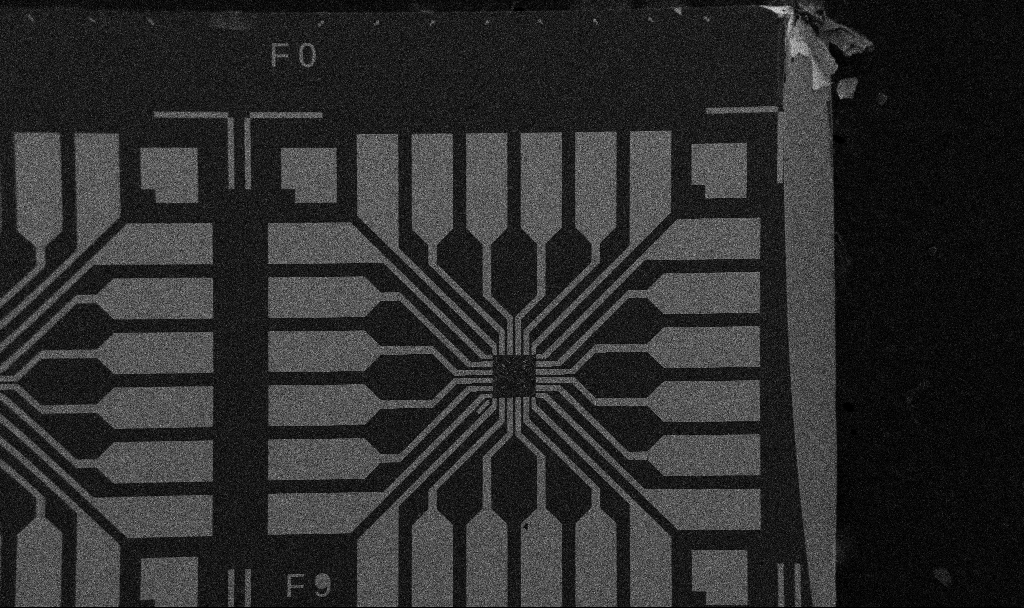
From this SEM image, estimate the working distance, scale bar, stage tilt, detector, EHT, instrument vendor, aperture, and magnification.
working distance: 10.7 mm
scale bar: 200000 nm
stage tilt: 0°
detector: SE2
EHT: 5 kV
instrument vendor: Zeiss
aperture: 30 µm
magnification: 0.1 K X